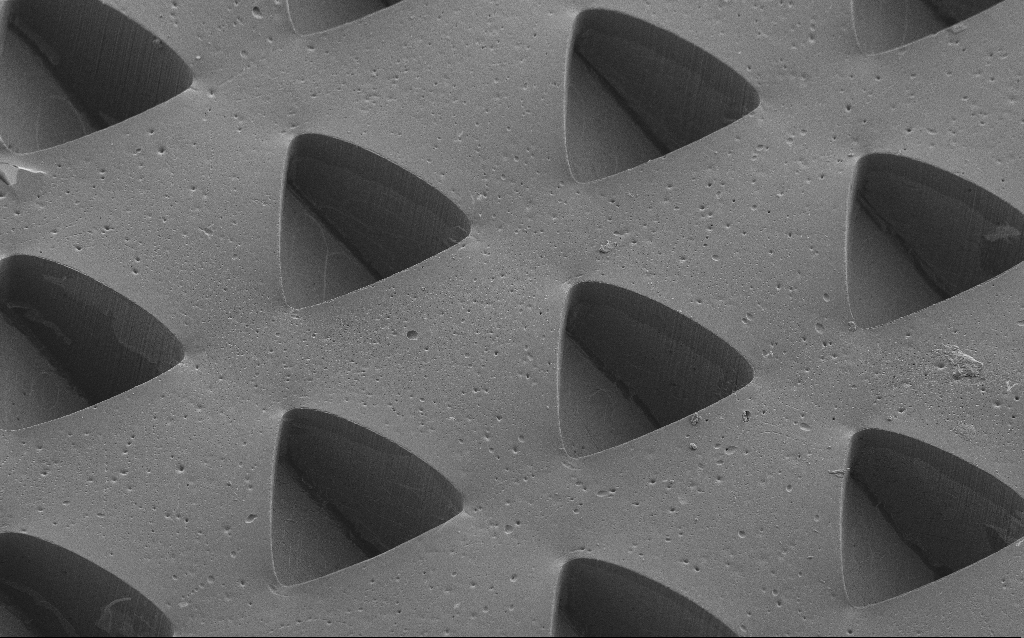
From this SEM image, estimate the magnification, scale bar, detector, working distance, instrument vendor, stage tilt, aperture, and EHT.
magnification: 0.272 K X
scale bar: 100000 nm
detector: SE2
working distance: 8 mm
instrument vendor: Zeiss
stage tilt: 35°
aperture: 30 µm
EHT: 5 kV